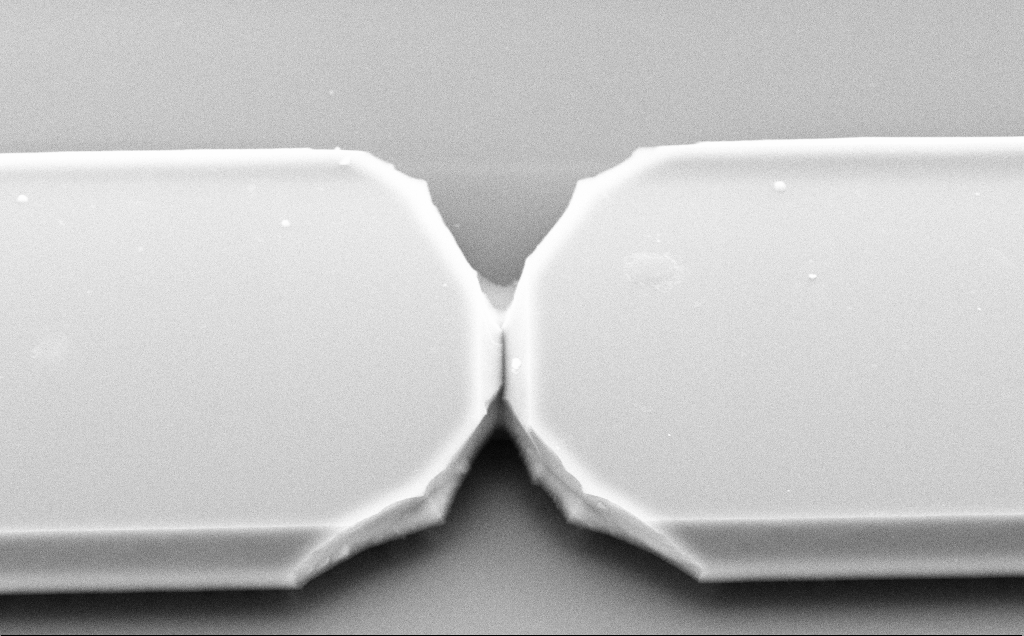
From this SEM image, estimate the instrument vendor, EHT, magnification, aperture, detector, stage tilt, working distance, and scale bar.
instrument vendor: Zeiss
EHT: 10 kV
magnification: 8.37 K X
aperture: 30 µm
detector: SE2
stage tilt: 31.9°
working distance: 10 mm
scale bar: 2000 nm